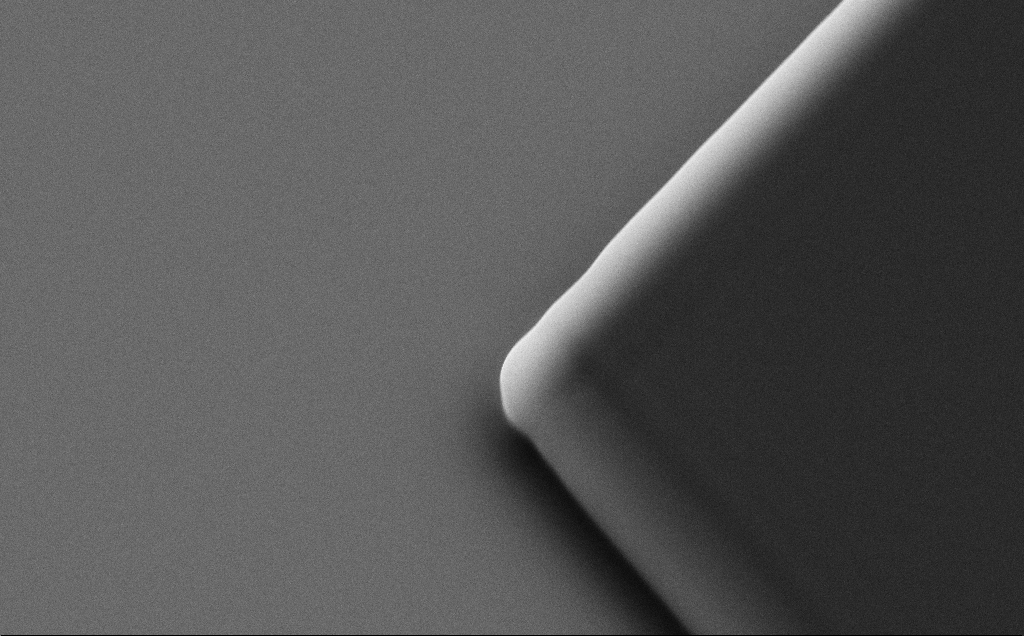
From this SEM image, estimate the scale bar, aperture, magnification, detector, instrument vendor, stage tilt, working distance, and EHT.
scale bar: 1000 nm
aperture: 30 µm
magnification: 16.47 K X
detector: SE2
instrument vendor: Zeiss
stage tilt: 35°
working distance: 8 mm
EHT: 10 kV